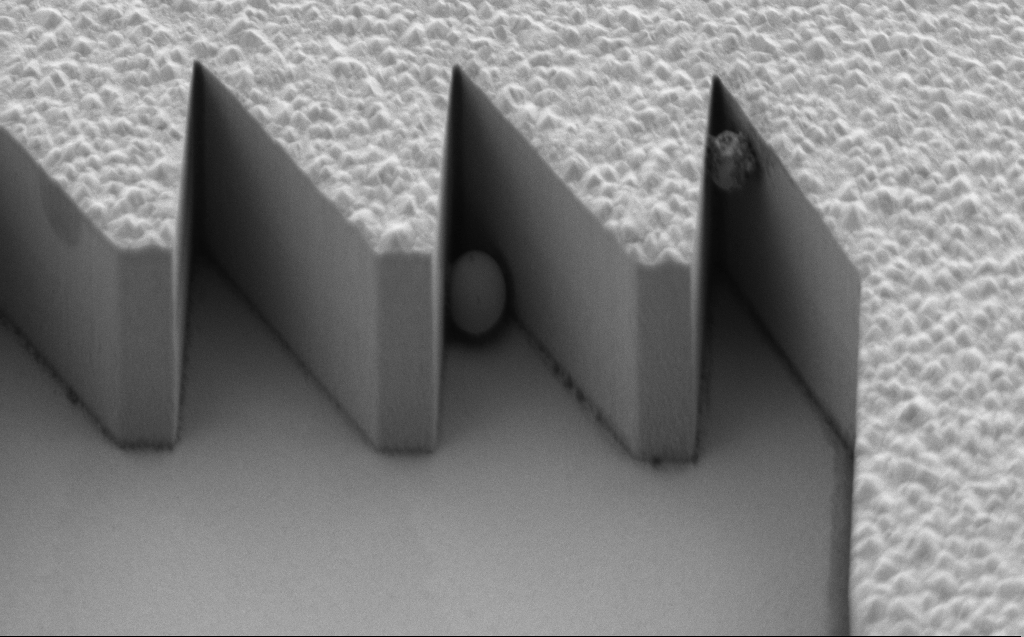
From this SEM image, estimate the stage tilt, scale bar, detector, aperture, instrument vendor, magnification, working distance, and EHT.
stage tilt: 45.1°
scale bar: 2000 nm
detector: SE2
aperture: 120 µm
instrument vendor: Zeiss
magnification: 8.3 K X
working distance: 7 mm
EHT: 3 kV